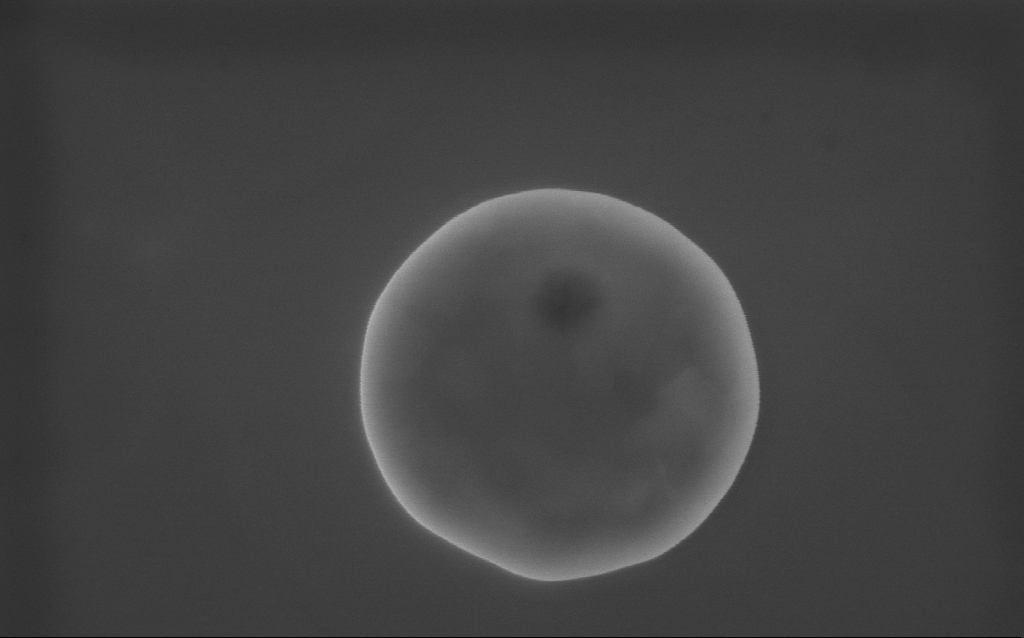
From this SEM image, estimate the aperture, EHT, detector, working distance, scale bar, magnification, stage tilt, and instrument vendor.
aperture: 30 µm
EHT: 10 kV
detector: InLens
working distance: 2 mm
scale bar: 200 nm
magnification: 115 K X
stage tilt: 0°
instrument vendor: Zeiss